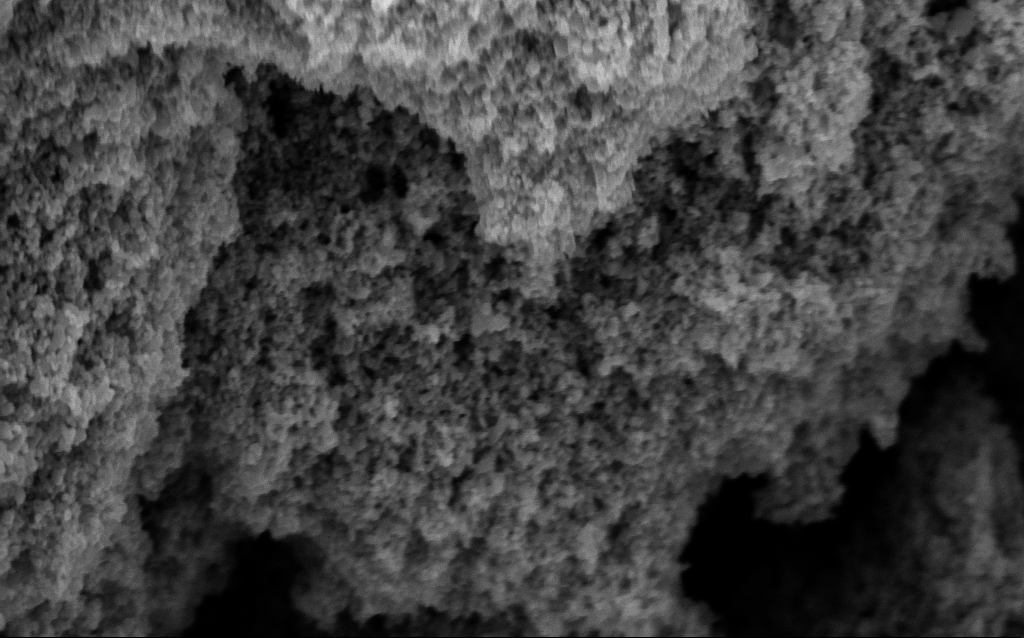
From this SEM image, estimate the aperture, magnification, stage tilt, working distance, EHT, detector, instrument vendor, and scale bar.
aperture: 30 µm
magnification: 76.33 K X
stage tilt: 0°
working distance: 9.6 mm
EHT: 5 kV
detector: InLens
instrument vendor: Zeiss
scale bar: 200 nm